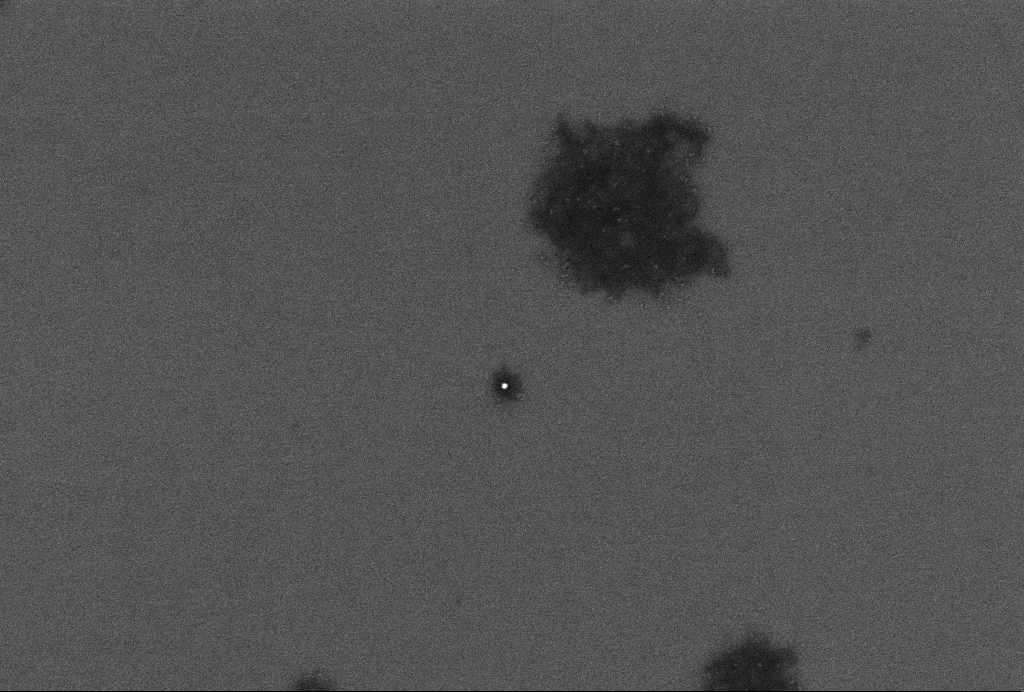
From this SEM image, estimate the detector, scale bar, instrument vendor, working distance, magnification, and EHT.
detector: InLens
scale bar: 200 nm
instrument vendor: Zeiss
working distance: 3.3 mm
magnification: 86.19 K X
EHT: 2 kV